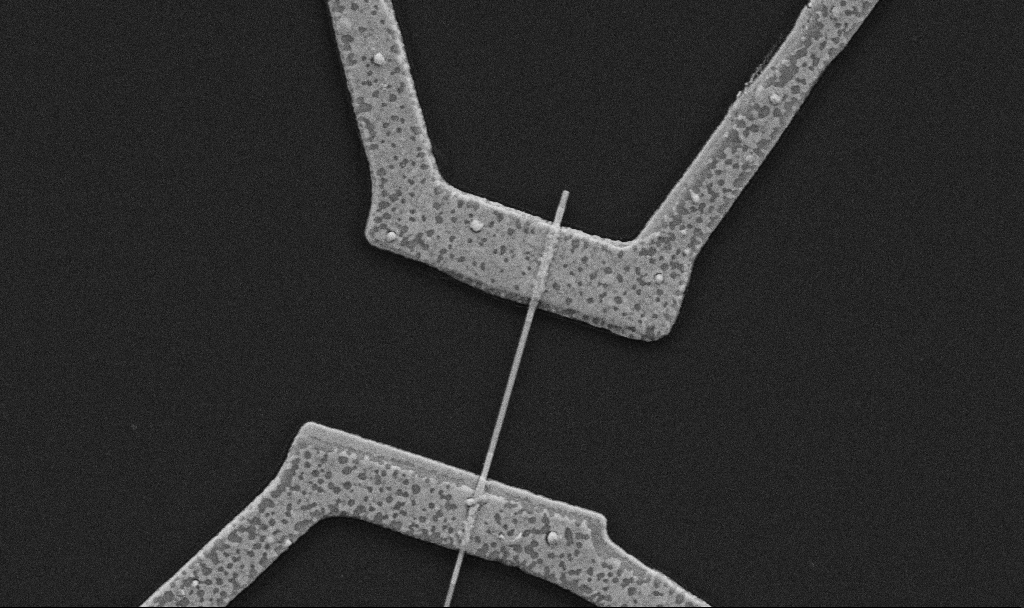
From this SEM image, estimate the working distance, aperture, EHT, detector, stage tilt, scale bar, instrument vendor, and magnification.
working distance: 10.7 mm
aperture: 30 µm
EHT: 5 kV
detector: SE2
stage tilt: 0°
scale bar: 1000 nm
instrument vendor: Zeiss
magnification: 30 K X